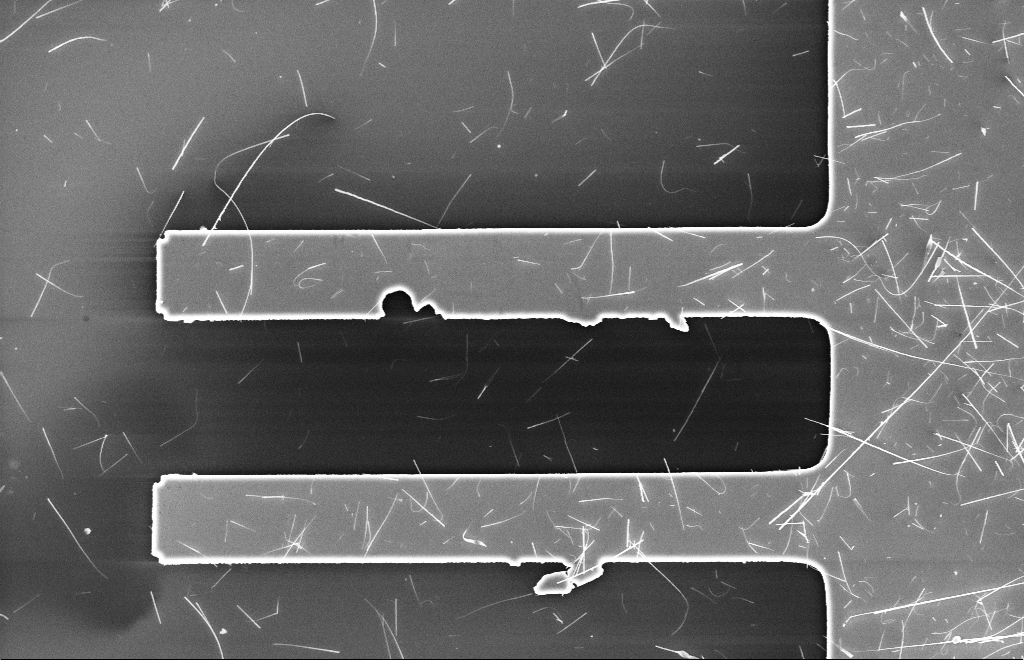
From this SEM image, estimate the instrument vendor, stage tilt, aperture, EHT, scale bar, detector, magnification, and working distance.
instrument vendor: Zeiss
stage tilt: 0°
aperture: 20 µm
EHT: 10 kV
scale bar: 10000 nm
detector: InLens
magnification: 2.5 K X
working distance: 6 mm